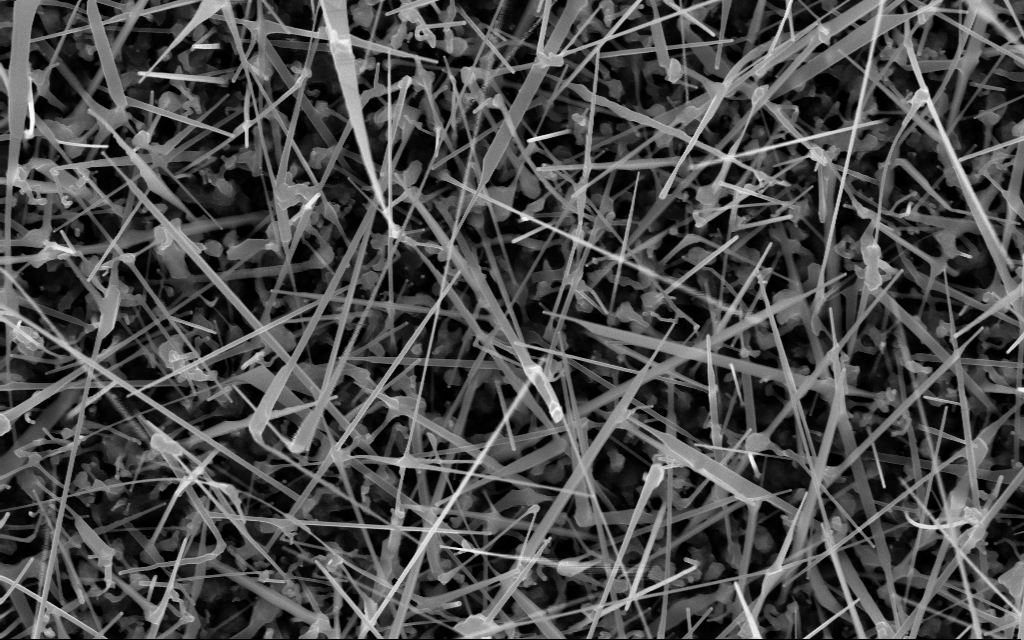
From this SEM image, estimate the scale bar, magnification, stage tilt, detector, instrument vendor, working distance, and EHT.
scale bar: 1000 nm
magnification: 40 K X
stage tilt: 0°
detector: InLens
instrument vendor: Zeiss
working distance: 7 mm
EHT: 10 kV